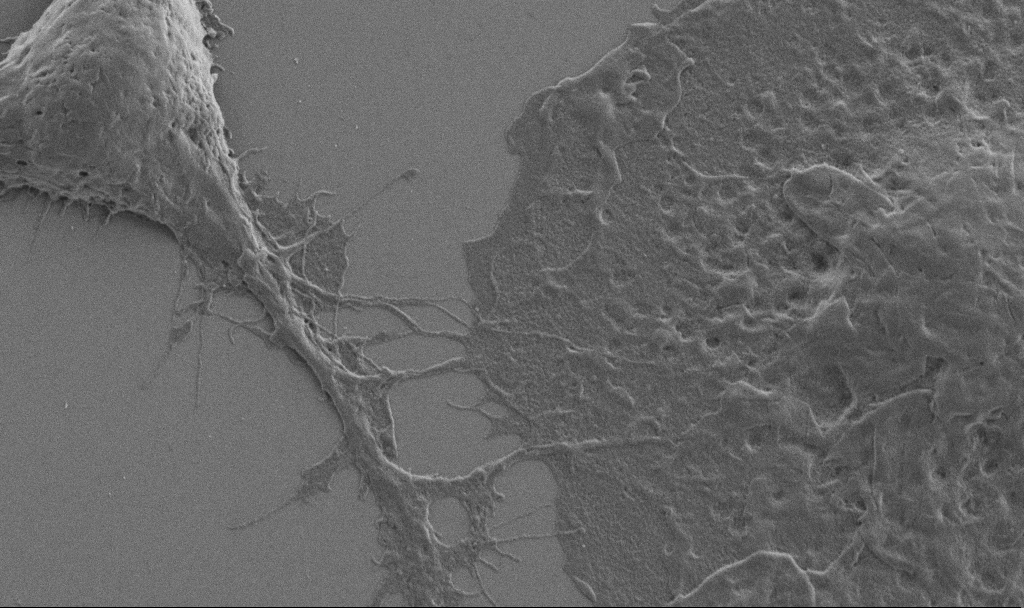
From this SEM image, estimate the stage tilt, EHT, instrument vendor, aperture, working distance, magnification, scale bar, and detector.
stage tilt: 0°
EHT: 1 kV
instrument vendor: Zeiss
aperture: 30 µm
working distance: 6.9 mm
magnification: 10 K X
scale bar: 2000 nm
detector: SE2